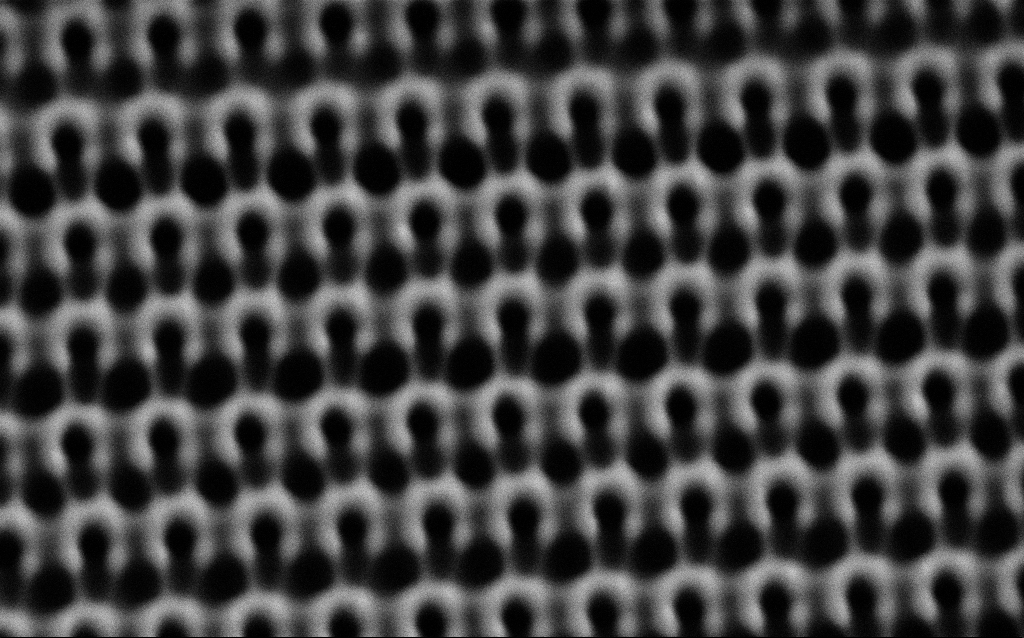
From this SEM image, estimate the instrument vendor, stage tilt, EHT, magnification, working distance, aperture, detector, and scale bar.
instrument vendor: Zeiss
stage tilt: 0°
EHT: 1.5 kV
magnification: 67.82 K X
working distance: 4.7 mm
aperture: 30 µm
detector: SE2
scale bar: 1000 nm